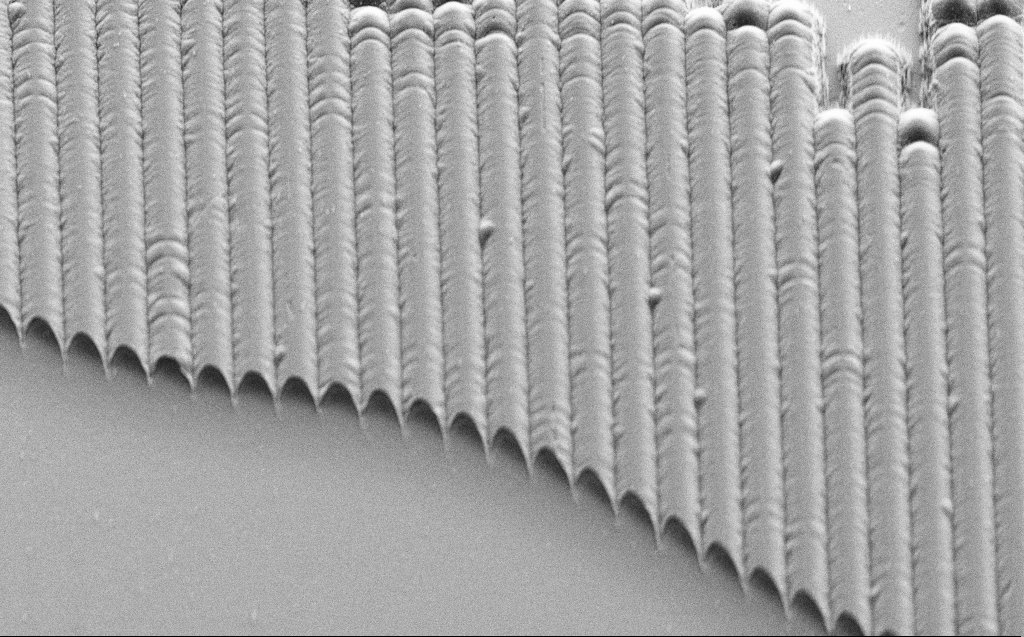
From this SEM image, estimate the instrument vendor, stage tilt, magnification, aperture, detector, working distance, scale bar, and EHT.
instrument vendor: Zeiss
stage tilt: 45°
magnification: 1.54 K X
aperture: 30 µm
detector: SE2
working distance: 9 mm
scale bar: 20000 nm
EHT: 3 kV